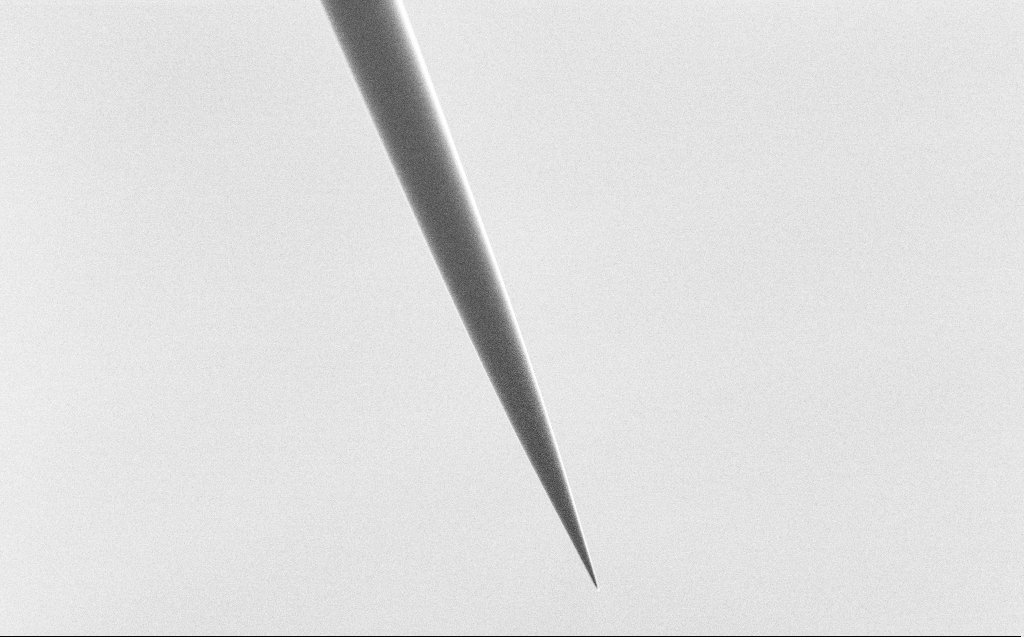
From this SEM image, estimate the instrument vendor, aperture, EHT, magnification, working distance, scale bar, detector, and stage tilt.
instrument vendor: Zeiss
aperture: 30 µm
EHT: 5 kV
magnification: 1 K X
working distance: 6 mm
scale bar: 20000 nm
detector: SE2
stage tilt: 45°